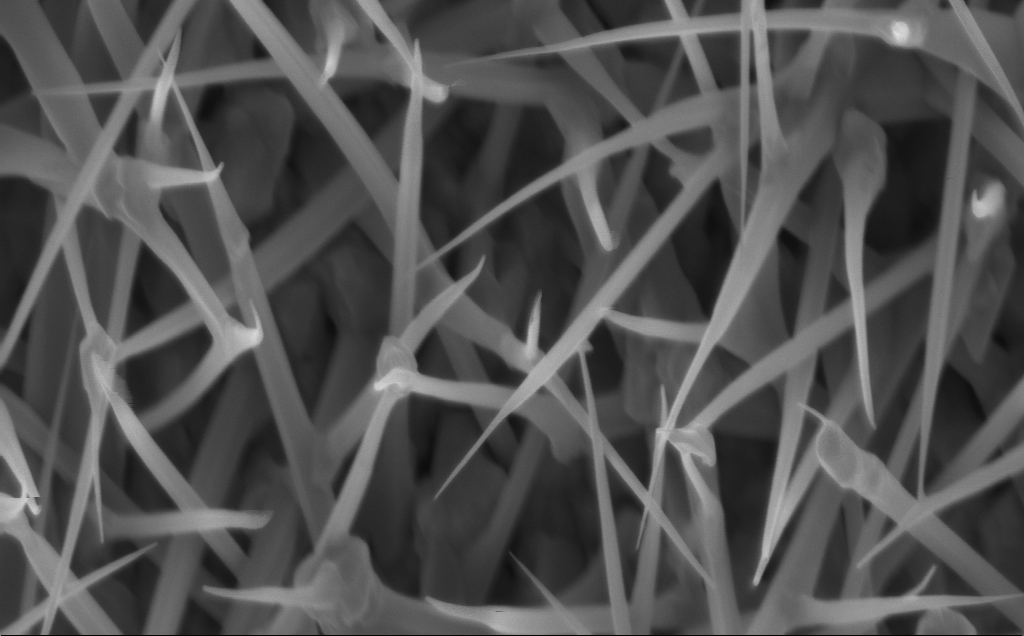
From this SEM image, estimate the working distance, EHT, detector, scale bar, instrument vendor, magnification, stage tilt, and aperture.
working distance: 5 mm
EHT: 5 kV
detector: InLens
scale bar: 200 nm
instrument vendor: Zeiss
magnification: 80 K X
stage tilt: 0°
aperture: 30 µm